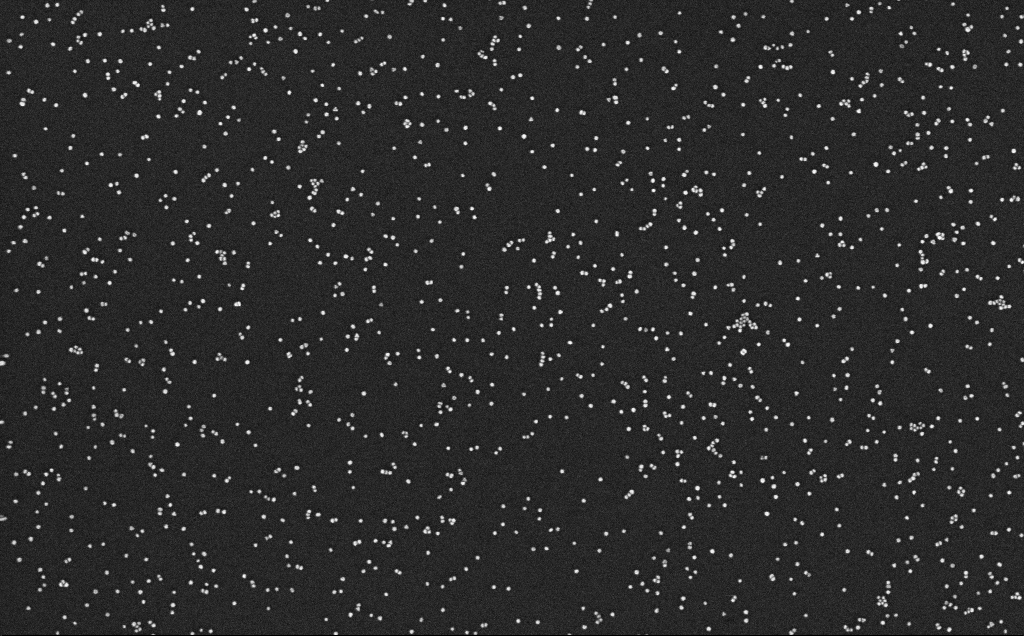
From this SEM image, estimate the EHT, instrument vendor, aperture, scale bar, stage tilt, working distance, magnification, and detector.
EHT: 10 kV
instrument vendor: Zeiss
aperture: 30 µm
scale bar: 200 nm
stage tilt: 0°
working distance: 3.1 mm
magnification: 100 K X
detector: InLens